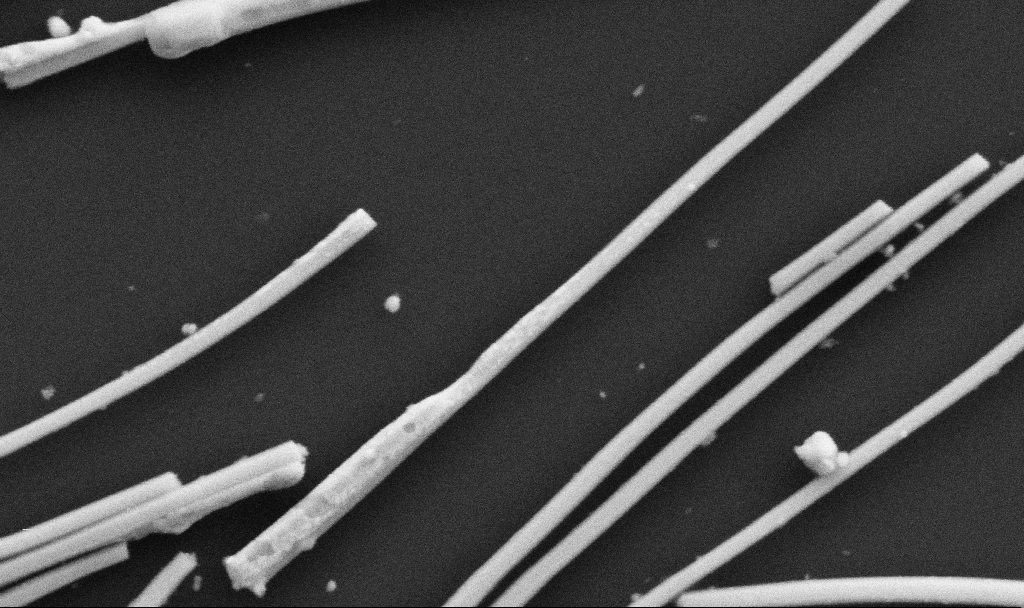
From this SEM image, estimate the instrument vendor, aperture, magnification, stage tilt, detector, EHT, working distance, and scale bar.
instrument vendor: Zeiss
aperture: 30 µm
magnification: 77.71 K X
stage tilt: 0°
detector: SE2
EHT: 5 kV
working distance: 10.7 mm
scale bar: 200 nm